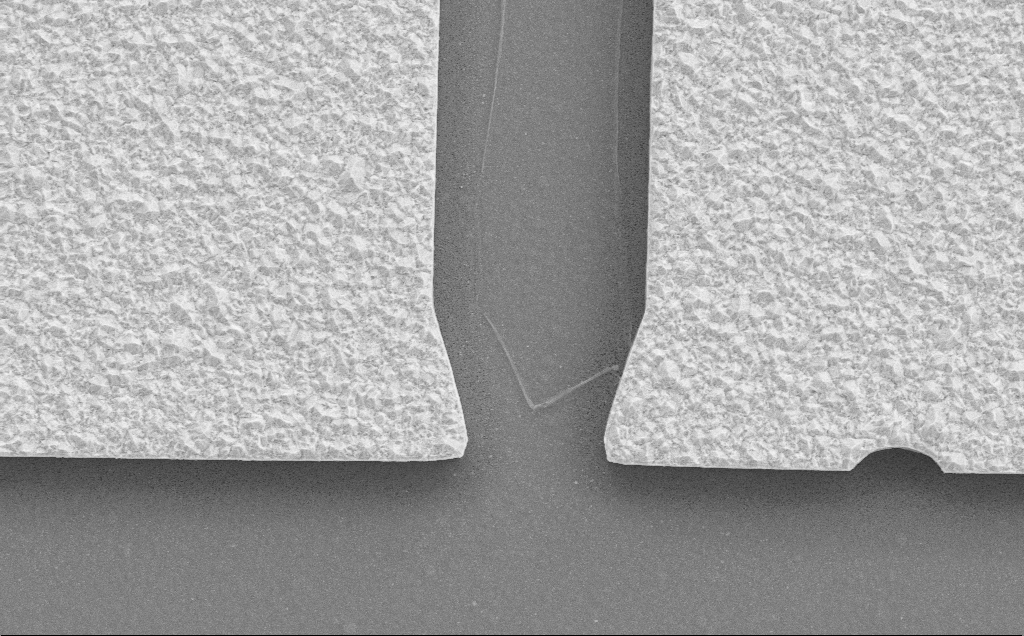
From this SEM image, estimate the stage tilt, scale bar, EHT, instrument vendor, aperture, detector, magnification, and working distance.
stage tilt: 0°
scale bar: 2000 nm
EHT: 10 kV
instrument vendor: Zeiss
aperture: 30 µm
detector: SE2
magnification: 7.64 K X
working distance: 9 mm